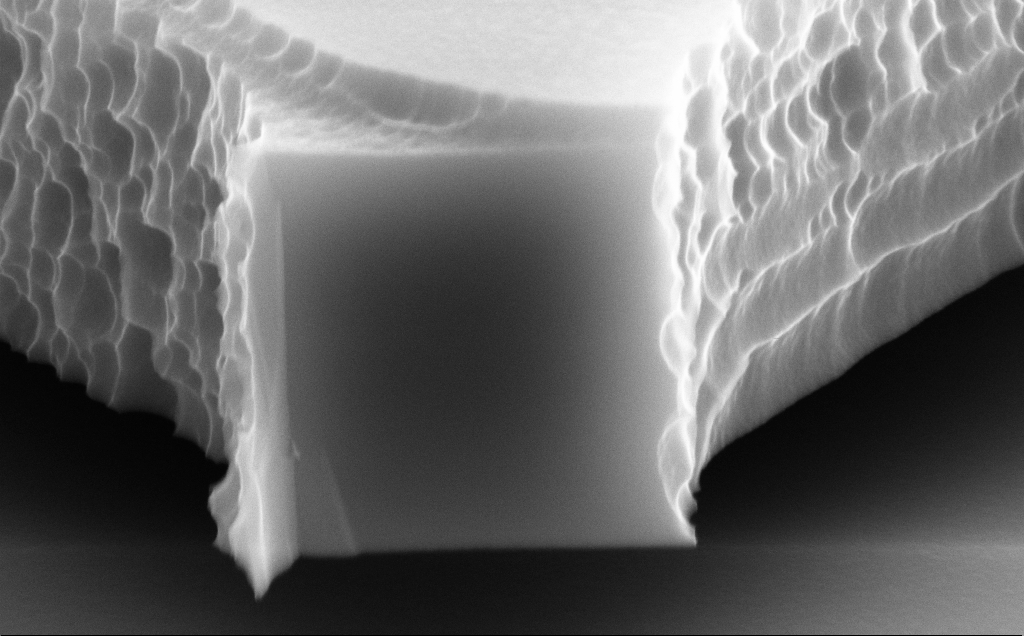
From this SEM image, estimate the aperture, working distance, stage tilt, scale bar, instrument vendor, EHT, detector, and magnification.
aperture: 30 µm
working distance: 9 mm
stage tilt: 70°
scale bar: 1000 nm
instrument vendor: Zeiss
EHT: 10 kV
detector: SE2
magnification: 69.6 K X